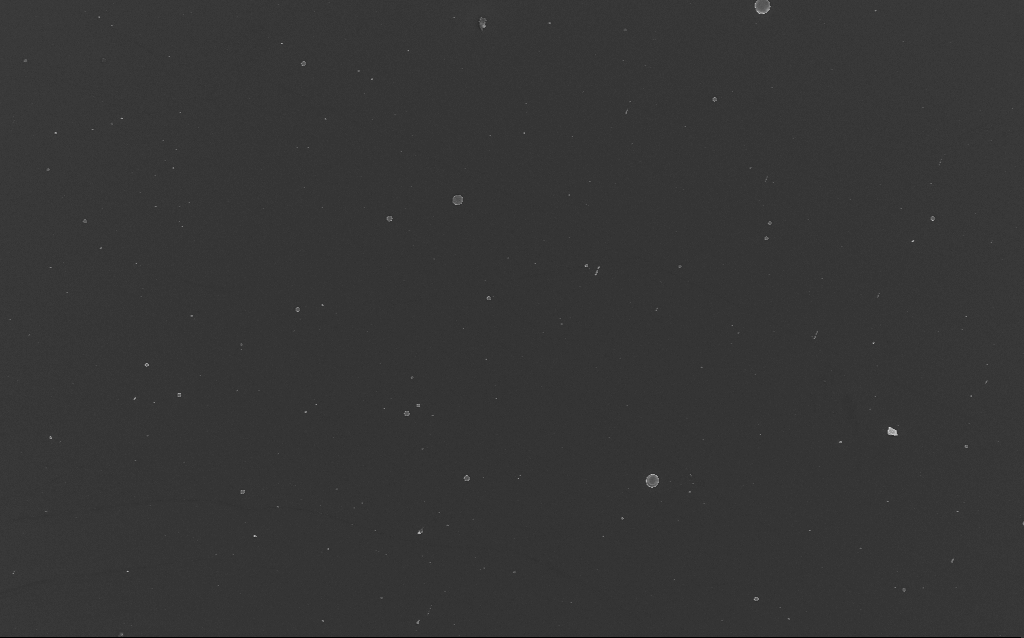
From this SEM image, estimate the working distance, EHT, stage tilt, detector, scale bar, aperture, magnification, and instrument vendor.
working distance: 3 mm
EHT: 10 kV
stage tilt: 0°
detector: InLens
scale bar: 20000 nm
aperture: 30 µm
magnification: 1.72 K X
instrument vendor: Zeiss